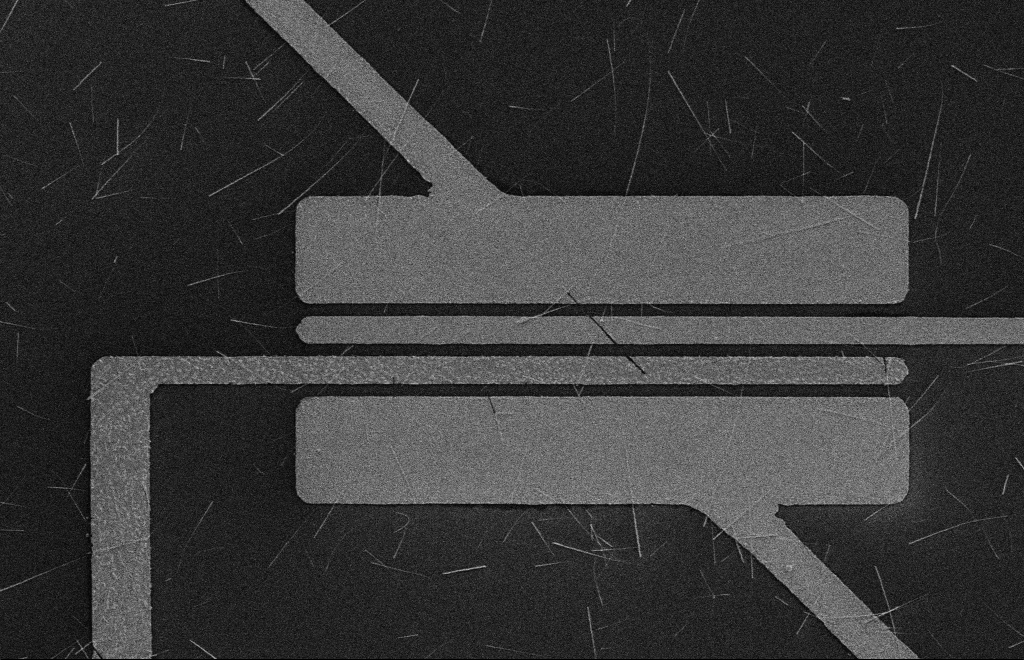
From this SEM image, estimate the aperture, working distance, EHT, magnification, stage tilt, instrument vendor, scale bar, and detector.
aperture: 10 µm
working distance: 16 mm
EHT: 5 kV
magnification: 3.7 K X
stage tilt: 0°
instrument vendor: Zeiss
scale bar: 10000 nm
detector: SE2